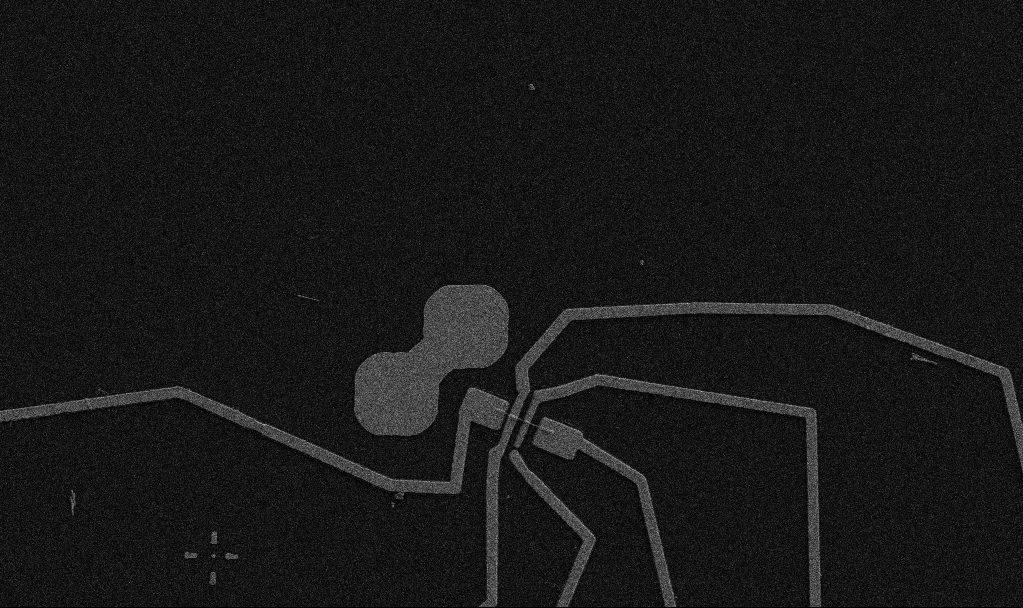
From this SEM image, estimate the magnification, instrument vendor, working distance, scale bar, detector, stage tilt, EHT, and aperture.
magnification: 5 K X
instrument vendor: Zeiss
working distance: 10.7 mm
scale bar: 10000 nm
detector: SE2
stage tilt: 0°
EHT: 5 kV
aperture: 30 µm